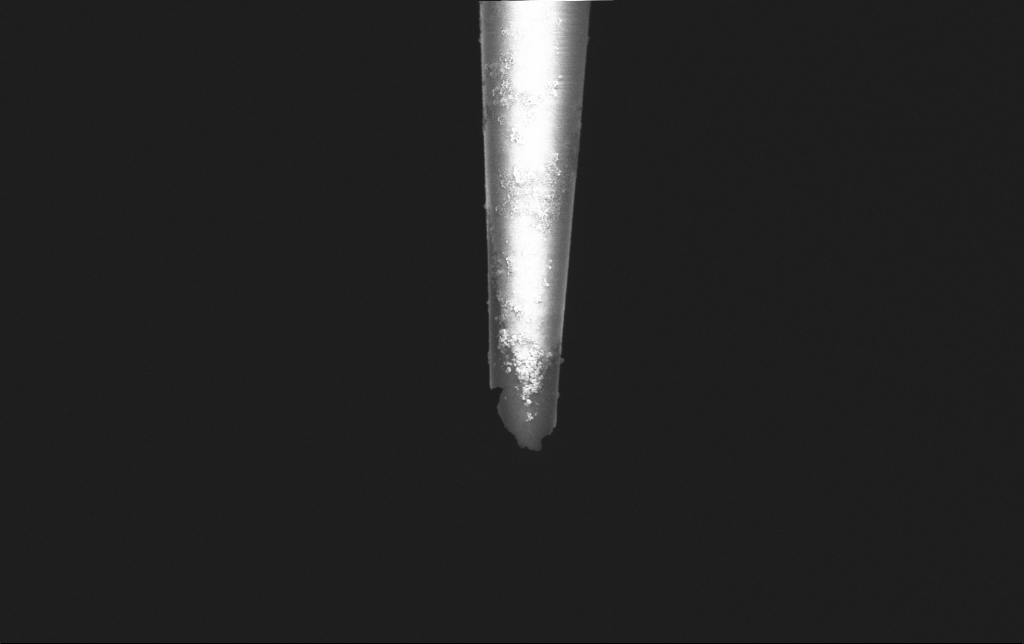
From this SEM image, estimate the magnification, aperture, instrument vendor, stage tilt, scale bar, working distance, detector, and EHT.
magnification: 25 K X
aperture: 30 µm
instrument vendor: Zeiss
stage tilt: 0°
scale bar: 2000 nm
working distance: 6 mm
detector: InLens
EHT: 2 kV